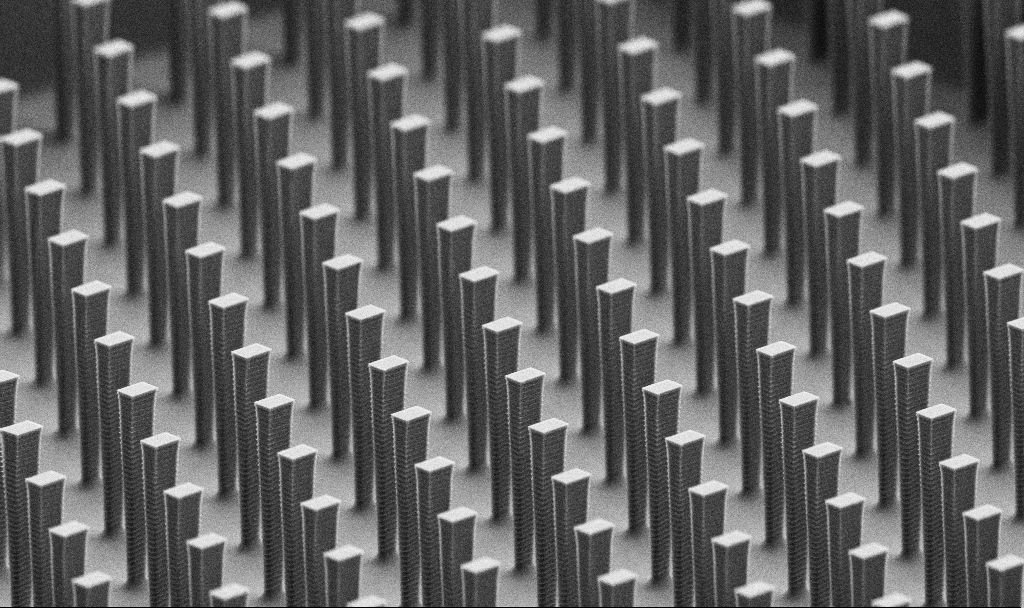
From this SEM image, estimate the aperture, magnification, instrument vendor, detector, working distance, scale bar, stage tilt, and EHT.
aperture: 30 µm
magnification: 4.8 K X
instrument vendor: Zeiss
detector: SE2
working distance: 6.2 mm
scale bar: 10000 nm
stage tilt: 70°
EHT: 5 kV